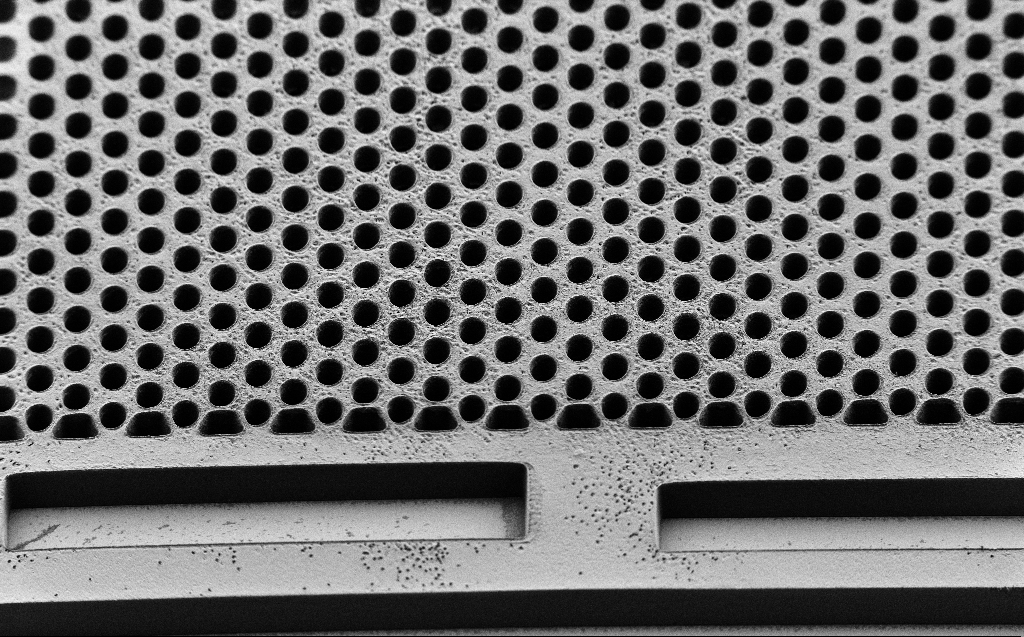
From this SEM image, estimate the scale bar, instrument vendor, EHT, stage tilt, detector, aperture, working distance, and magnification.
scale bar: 100000 nm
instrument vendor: Zeiss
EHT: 2 kV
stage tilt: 45°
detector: SE2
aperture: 30 µm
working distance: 7 mm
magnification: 0.311 K X